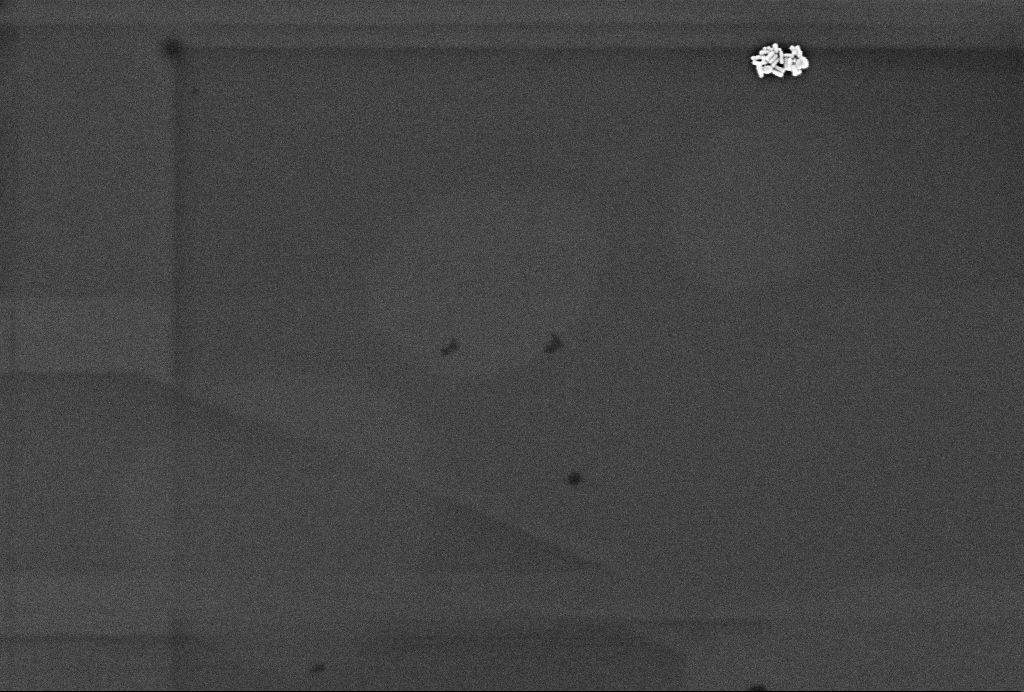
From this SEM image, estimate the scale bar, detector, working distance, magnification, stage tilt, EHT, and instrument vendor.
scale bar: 200 nm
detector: InLens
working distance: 3.3 mm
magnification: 60.84 K X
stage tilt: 0°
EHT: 2 kV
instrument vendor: Zeiss